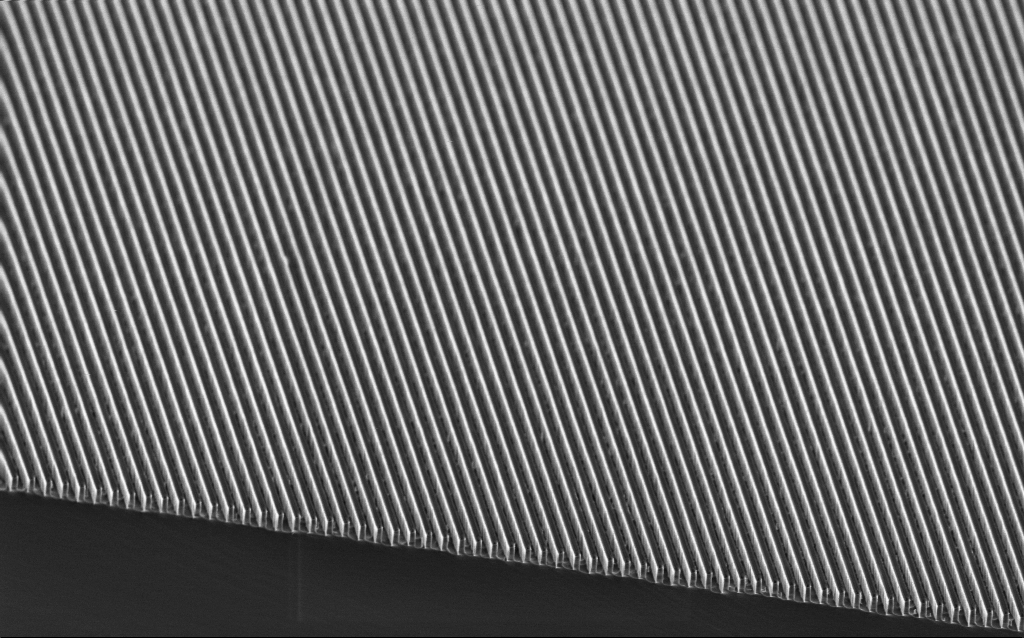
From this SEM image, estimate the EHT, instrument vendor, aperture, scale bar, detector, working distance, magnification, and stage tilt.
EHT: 2 kV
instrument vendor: Zeiss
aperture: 30 µm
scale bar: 2000 nm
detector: InLens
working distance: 3.6 mm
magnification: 10.97 K X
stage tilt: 45°